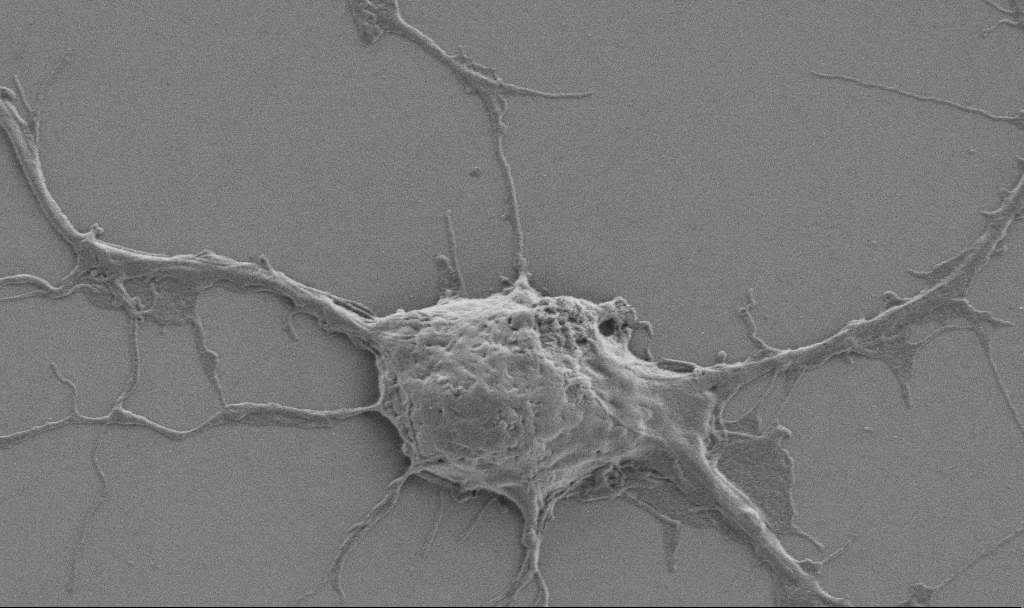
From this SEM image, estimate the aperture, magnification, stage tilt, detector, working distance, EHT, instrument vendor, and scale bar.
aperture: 30 µm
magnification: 10 K X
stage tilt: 0°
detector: SE2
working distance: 6.9 mm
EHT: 0.9 kV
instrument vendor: Zeiss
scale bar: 2000 nm